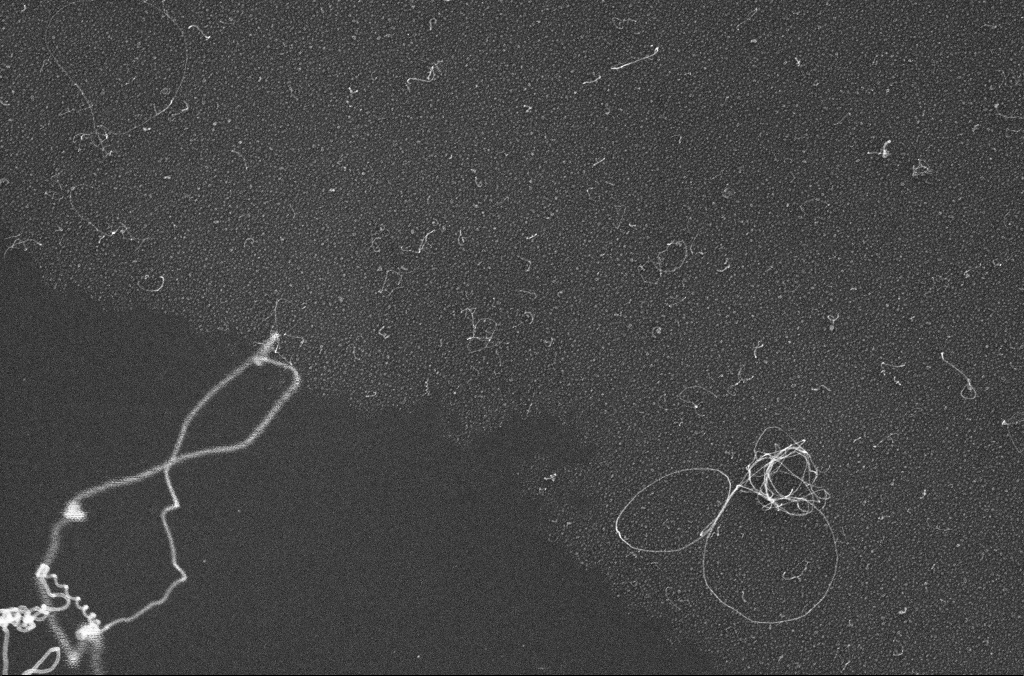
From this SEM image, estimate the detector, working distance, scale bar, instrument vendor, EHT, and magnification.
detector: InLens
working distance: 3 mm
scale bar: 1000 nm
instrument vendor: Zeiss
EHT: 10 kV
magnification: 50 K X